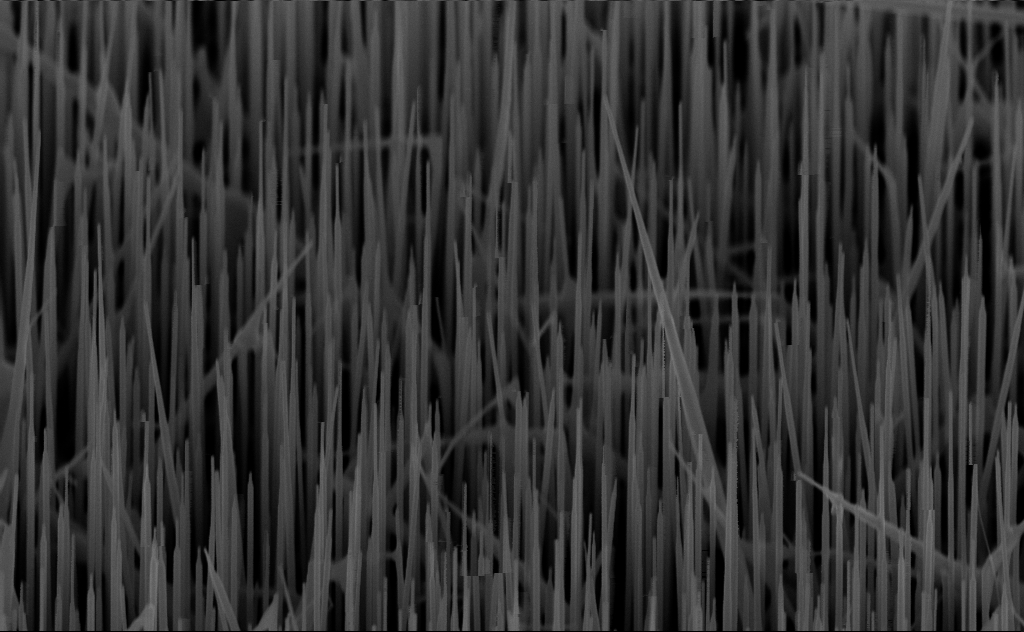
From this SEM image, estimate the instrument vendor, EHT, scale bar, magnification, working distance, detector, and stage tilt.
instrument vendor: Zeiss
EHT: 10 kV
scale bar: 1000 nm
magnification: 40 K X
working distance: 6 mm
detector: InLens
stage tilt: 44.2°